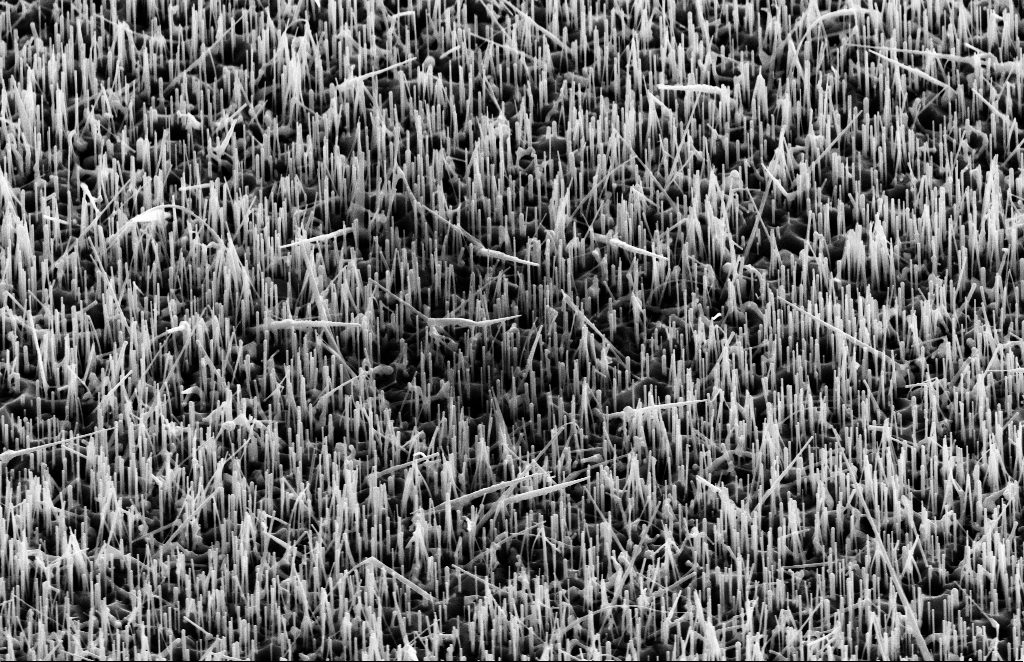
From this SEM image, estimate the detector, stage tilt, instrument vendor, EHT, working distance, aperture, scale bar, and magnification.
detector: SE2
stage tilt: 45°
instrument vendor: Zeiss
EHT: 10 kV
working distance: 15 mm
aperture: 20 µm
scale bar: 1000 nm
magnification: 20 K X